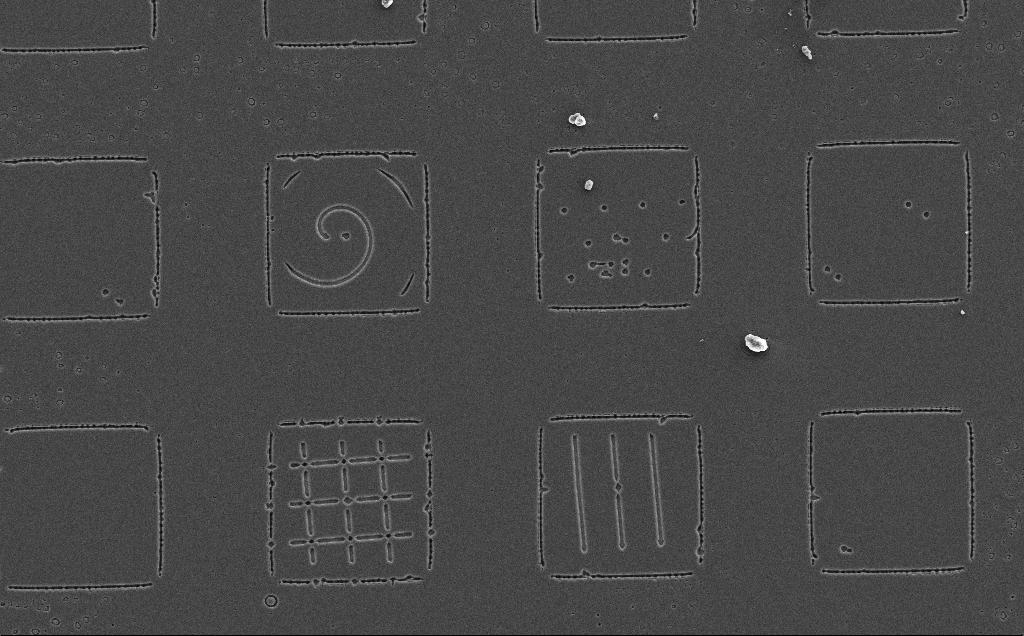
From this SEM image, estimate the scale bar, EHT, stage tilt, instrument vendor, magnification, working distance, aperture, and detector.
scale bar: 100000 nm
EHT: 10 kV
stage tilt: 0°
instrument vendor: Zeiss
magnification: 0.705 K X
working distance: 13 mm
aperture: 30 µm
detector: SE2